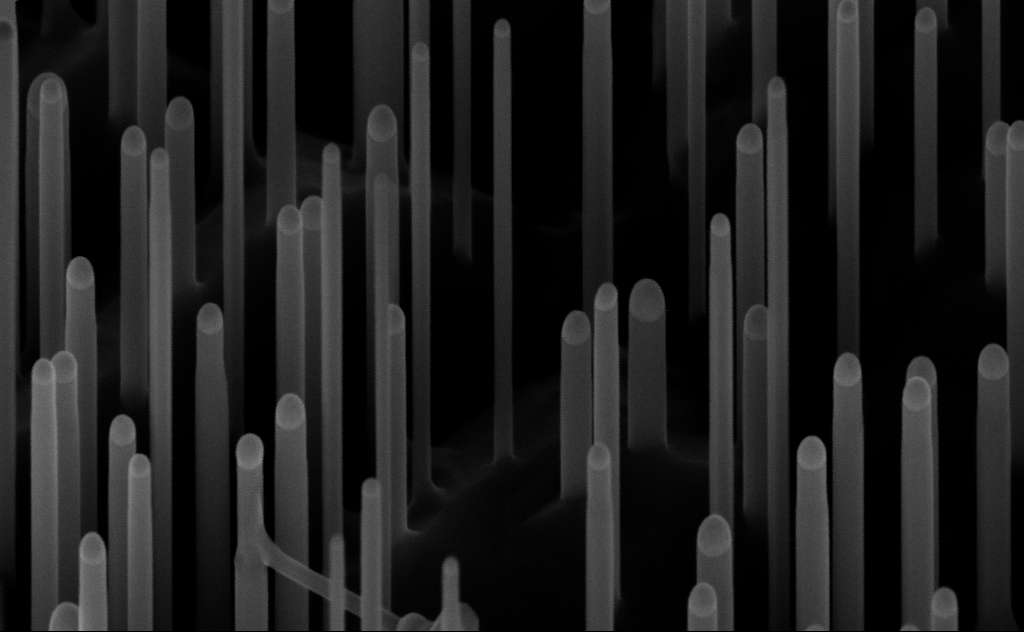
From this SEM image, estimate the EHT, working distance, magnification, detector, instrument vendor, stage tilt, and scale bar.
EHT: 10 kV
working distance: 7 mm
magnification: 150 K X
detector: InLens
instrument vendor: Zeiss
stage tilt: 45°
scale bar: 200 nm